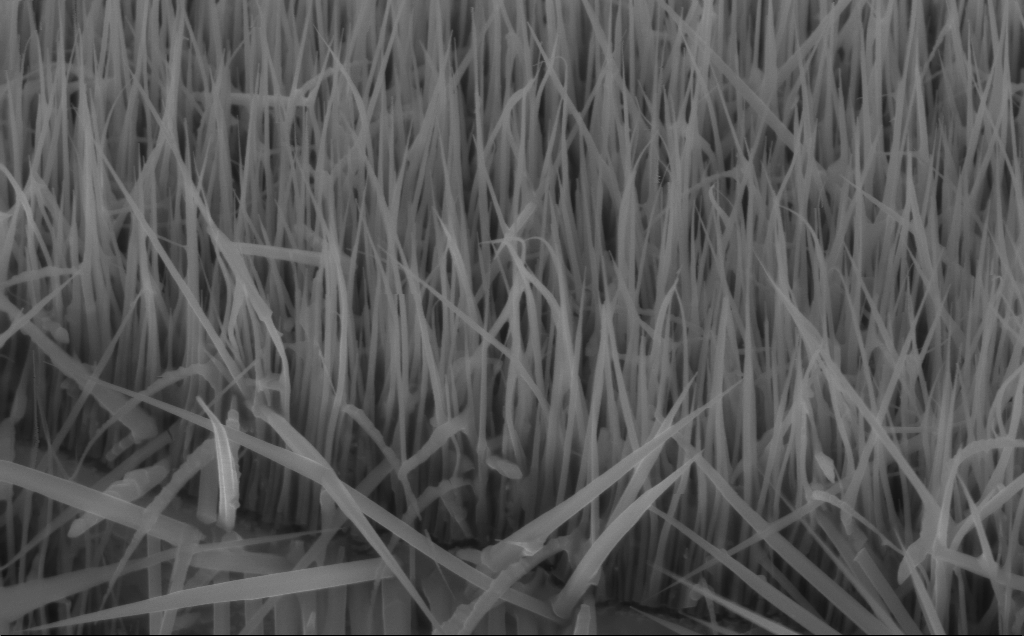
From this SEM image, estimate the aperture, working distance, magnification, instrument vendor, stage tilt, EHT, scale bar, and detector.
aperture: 30 µm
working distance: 5 mm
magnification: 50.87 K X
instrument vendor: Zeiss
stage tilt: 45°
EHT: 10 kV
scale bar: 1000 nm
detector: InLens